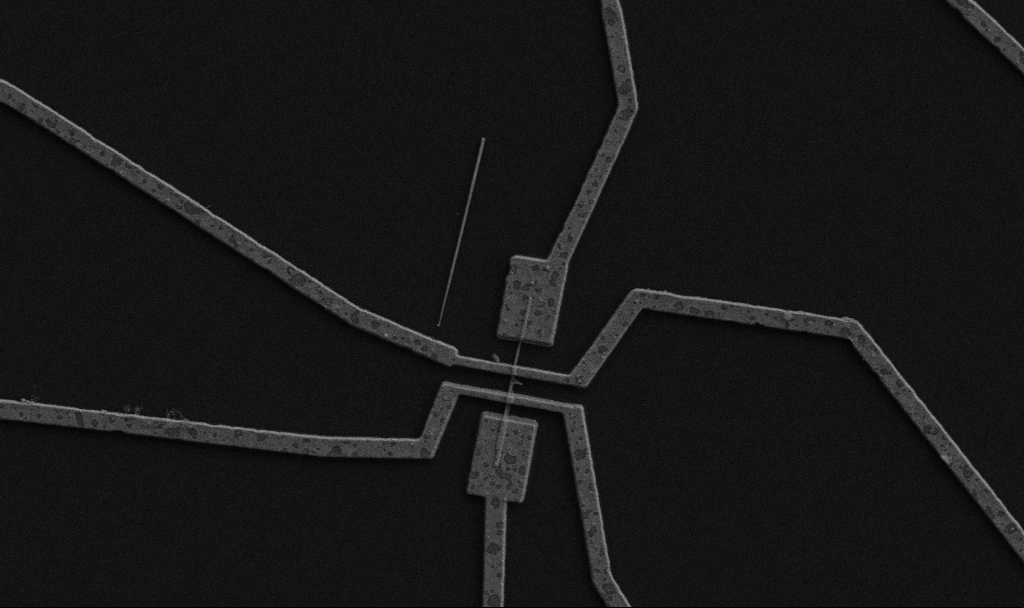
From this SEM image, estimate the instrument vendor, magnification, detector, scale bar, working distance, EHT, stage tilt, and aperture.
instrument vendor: Zeiss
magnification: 10 K X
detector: SE2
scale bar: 2000 nm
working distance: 9.7 mm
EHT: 5 kV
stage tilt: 0°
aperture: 30 µm